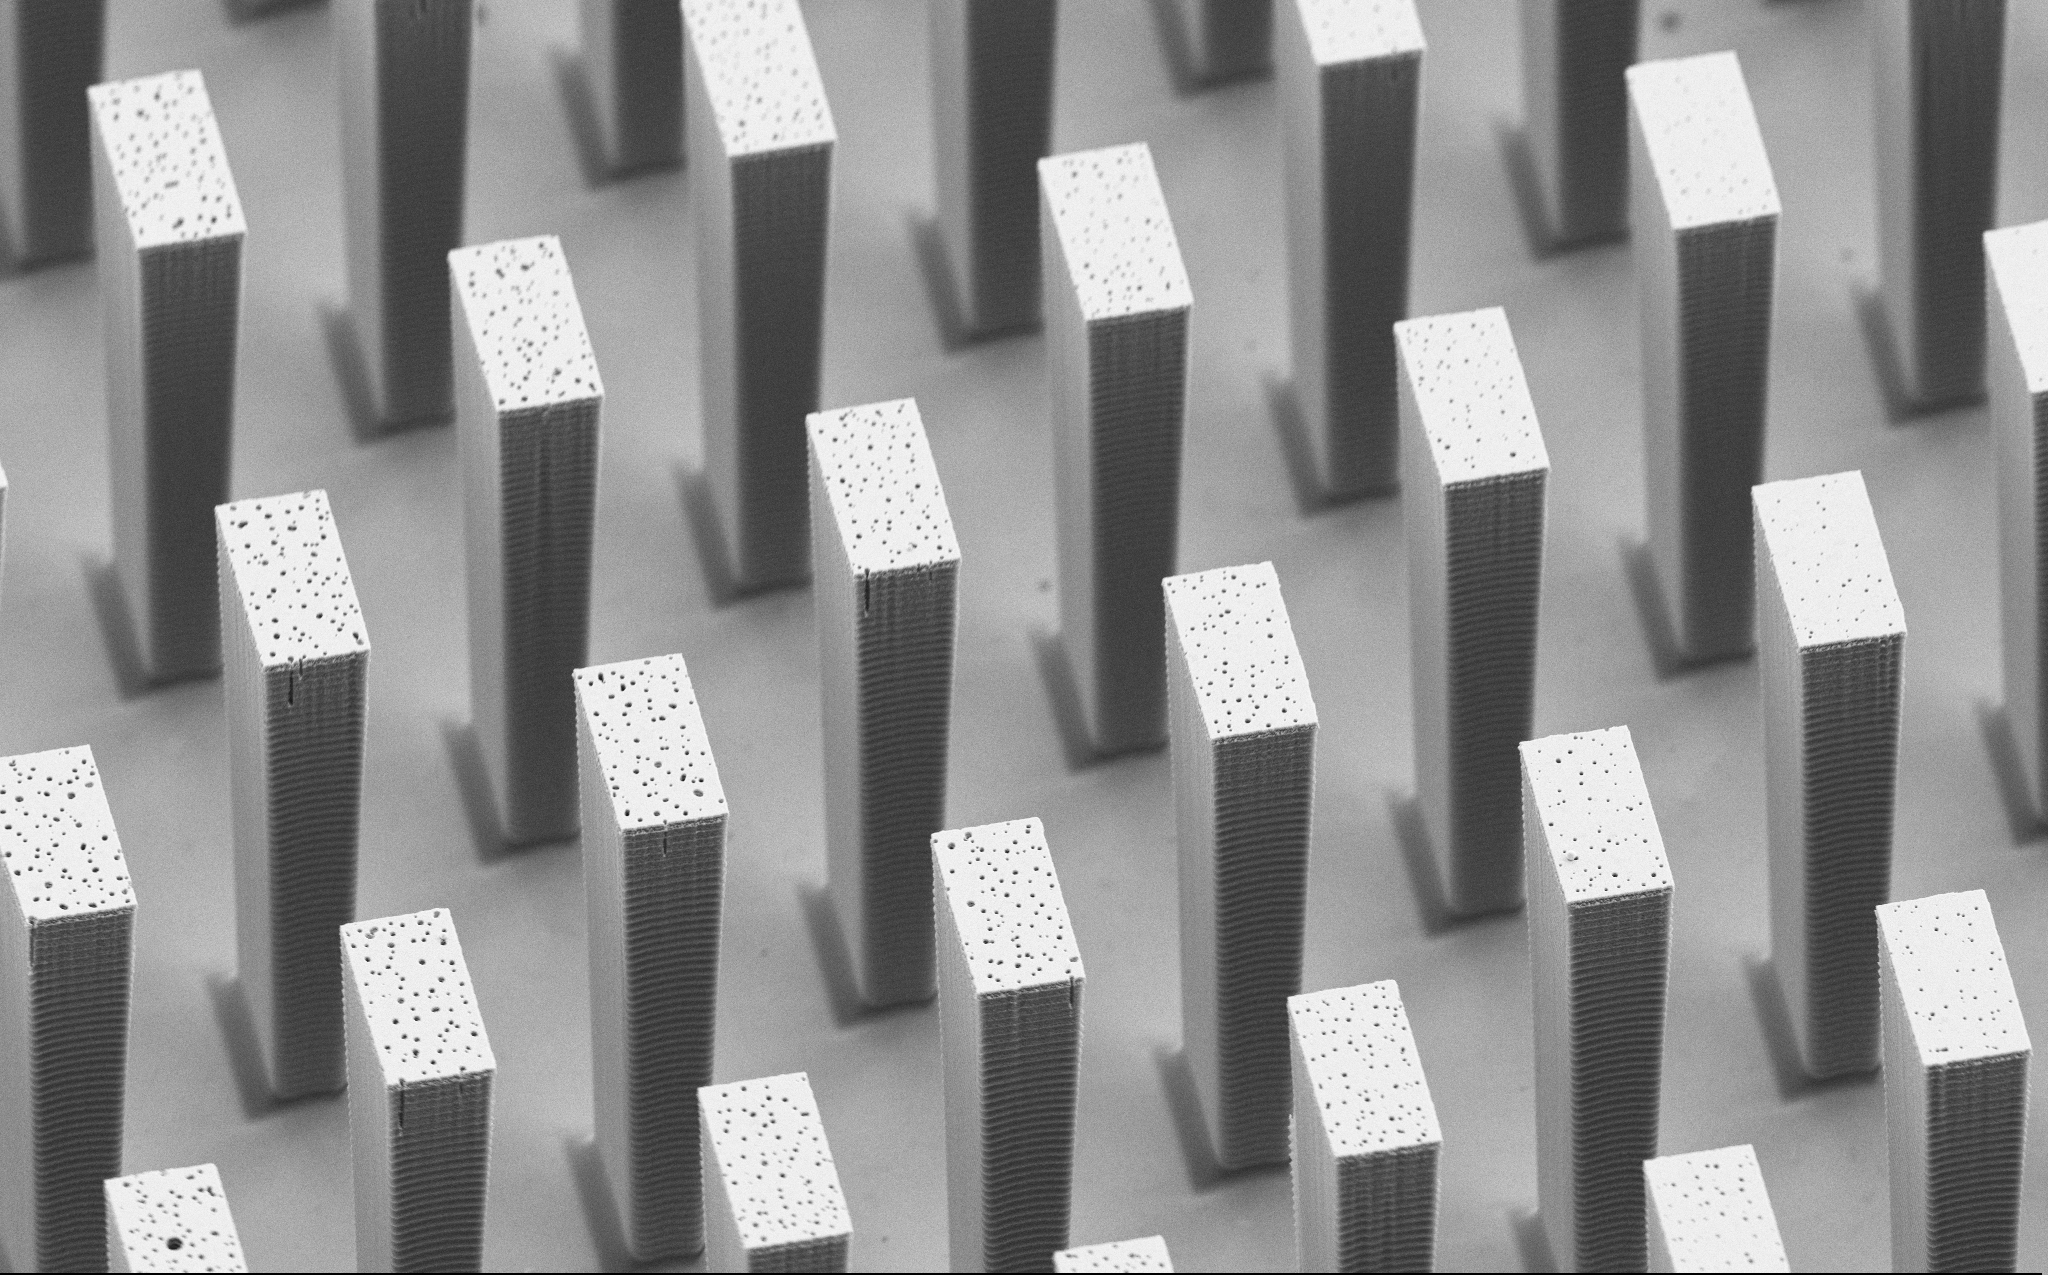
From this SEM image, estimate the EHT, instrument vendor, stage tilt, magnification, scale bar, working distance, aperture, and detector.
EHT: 5 kV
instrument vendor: Zeiss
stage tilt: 45°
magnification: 5.5 K X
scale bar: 10000 nm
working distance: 8 mm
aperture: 30 µm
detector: SE2